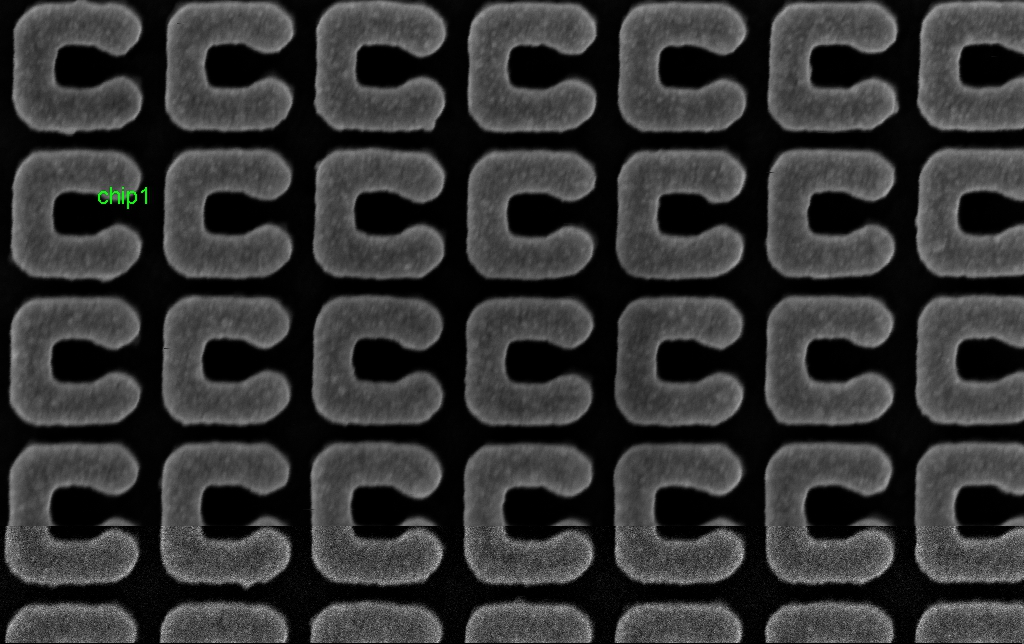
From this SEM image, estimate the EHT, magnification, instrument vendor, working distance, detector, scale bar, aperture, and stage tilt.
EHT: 5 kV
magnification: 121.03 K X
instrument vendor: Zeiss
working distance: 3.1 mm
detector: InLens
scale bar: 200 nm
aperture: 30 µm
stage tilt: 0°